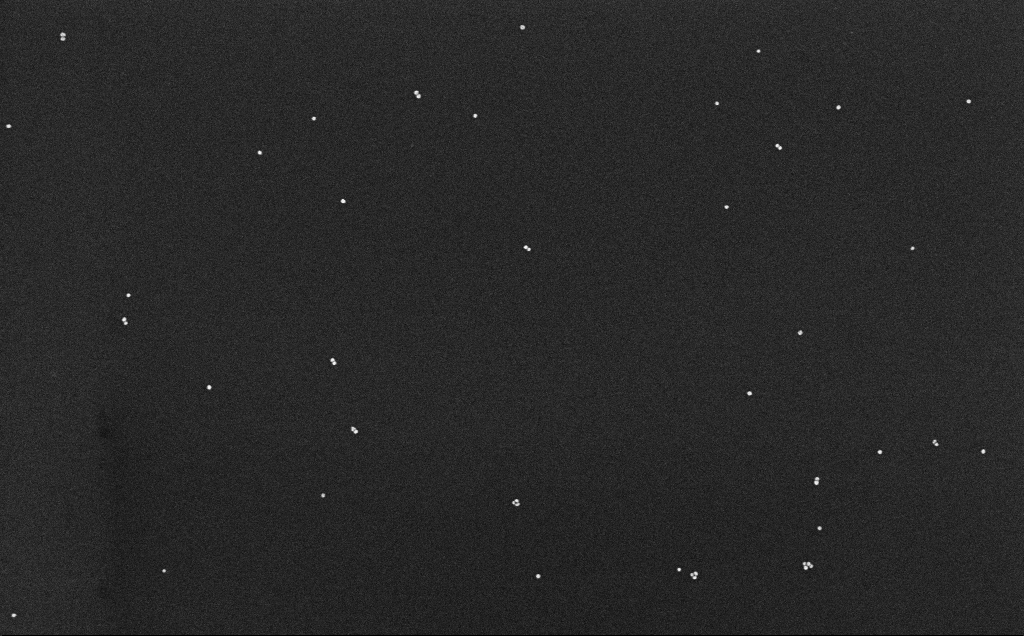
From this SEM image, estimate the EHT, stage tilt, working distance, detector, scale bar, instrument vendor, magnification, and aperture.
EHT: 10 kV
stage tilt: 0°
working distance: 6.6 mm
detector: InLens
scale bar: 200 nm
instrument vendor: Zeiss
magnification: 100 K X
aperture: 30 µm